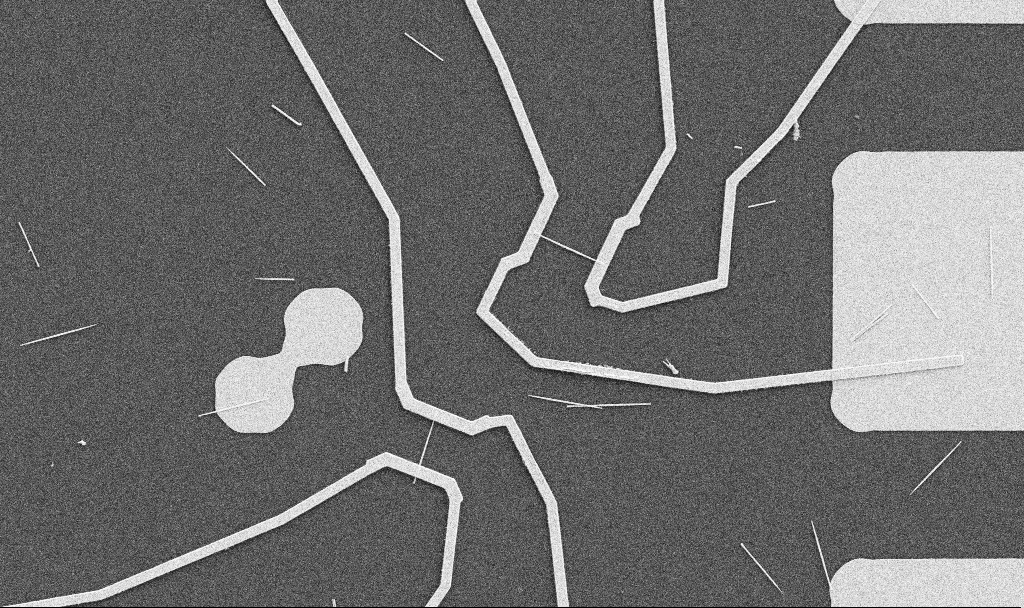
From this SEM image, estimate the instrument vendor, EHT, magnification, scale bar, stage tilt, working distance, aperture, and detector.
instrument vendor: Zeiss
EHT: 5 kV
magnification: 5 K X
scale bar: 10000 nm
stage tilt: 0°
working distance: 10.7 mm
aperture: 30 µm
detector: SE2